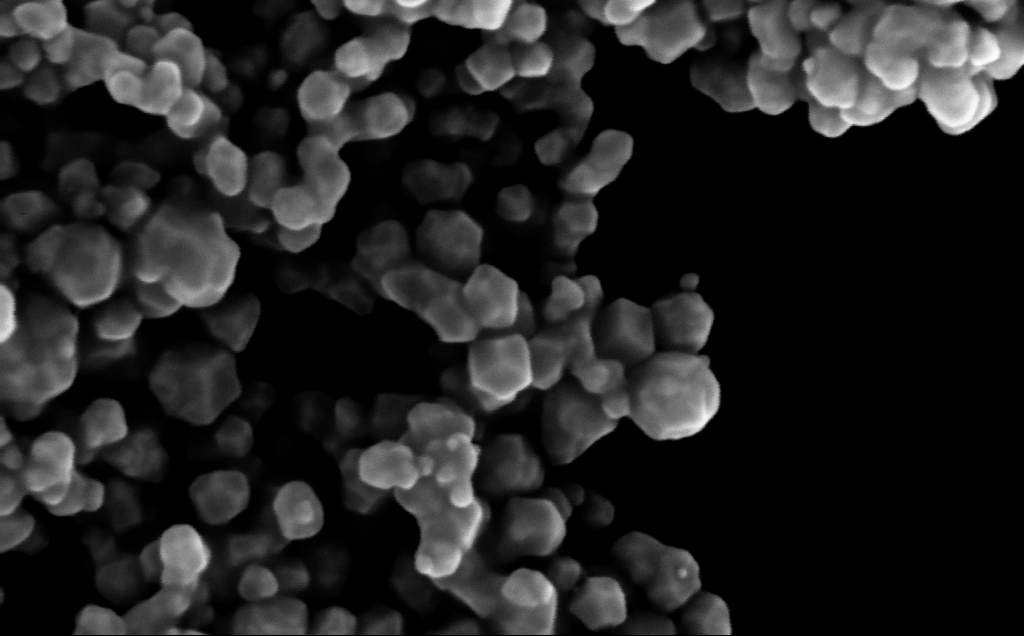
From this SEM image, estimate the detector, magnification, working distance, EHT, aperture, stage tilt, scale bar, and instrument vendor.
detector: InLens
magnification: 371.33 K X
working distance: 4 mm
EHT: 10 kV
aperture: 30 µm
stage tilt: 0°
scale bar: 100 nm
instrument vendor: Zeiss